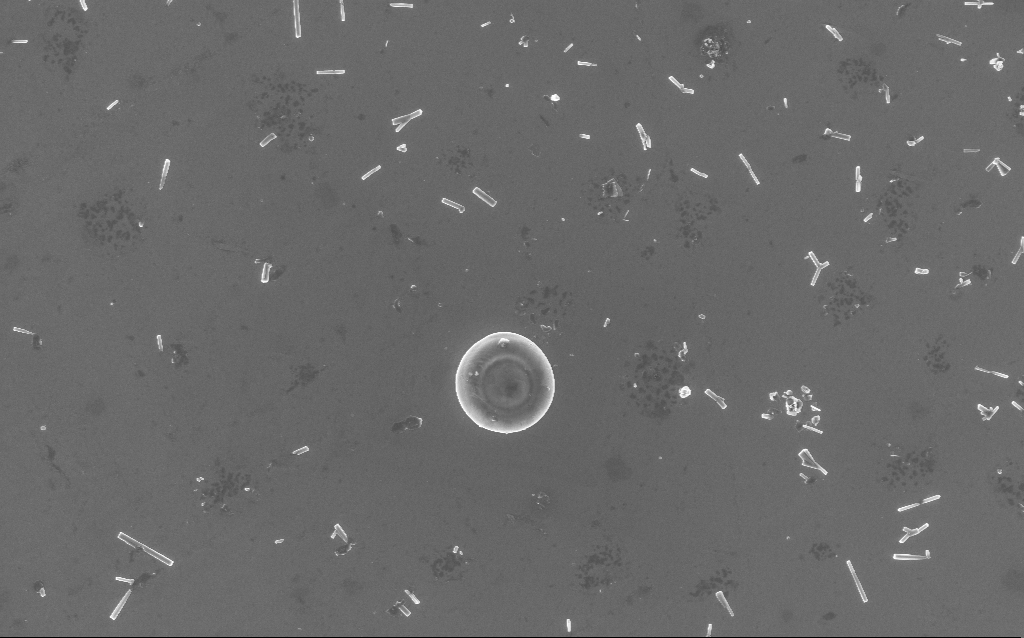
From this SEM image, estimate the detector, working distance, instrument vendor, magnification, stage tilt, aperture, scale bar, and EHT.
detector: InLens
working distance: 3 mm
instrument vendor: Zeiss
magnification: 9.78 K X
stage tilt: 0°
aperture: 30 µm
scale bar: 2000 nm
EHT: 5 kV